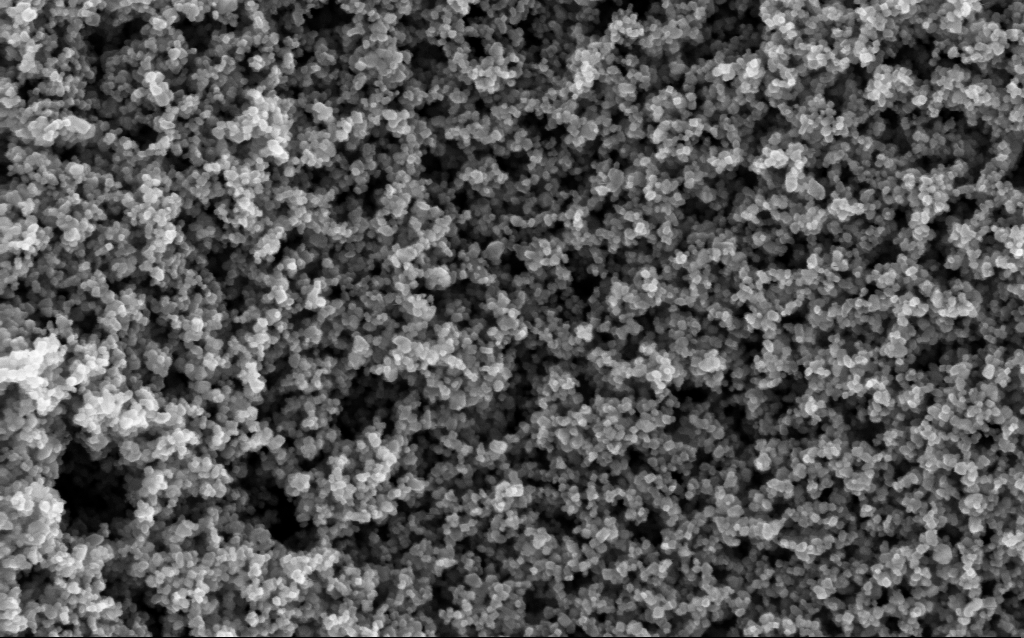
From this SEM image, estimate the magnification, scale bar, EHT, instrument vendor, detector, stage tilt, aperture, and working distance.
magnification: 130 K X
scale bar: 100 nm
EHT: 5 kV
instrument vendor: Zeiss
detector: InLens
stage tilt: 0°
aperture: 30 µm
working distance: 5.3 mm